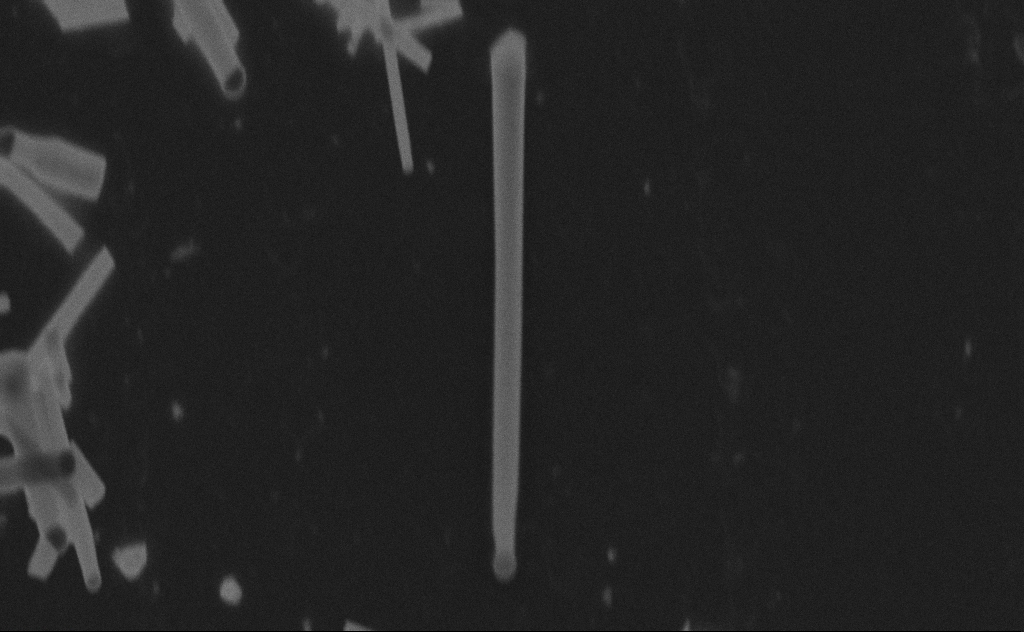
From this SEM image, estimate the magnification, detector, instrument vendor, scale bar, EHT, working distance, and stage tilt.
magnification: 112.65 K X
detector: SE2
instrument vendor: Zeiss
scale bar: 200 nm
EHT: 20 kV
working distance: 9 mm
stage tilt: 0°